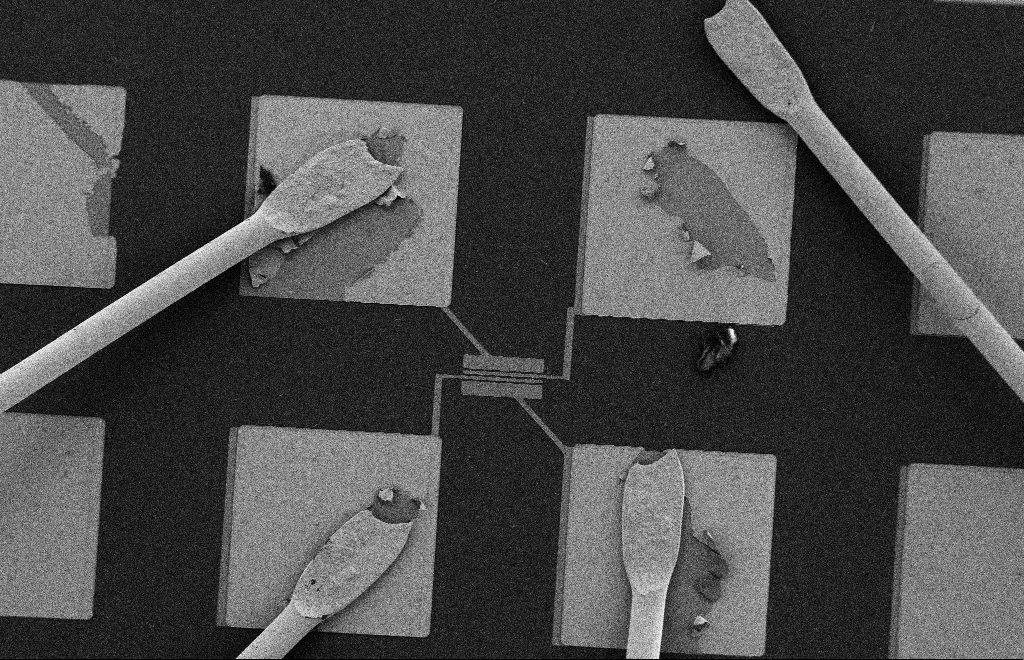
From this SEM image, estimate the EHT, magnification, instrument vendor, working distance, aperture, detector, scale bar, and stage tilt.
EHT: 2 kV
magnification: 0.487 K X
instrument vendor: Zeiss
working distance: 10 mm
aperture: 20 µm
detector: SE2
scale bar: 20000 nm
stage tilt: -0.3°